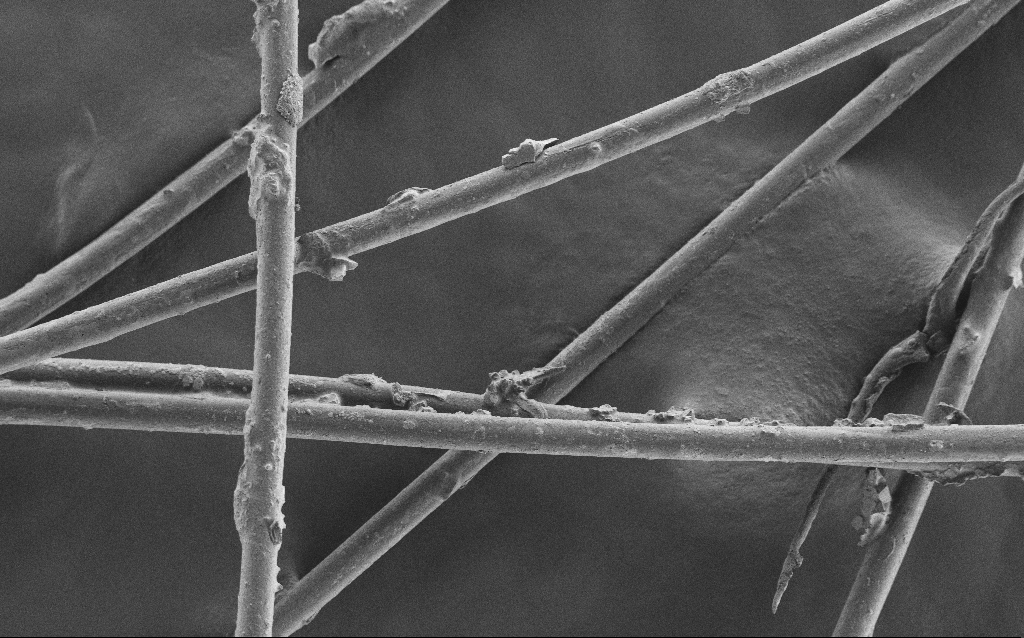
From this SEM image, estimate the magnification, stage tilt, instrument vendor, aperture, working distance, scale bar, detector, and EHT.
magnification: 0.567 K X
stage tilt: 0°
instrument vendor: Zeiss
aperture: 30 µm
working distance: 5 mm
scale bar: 100000 nm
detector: SE2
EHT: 1 kV